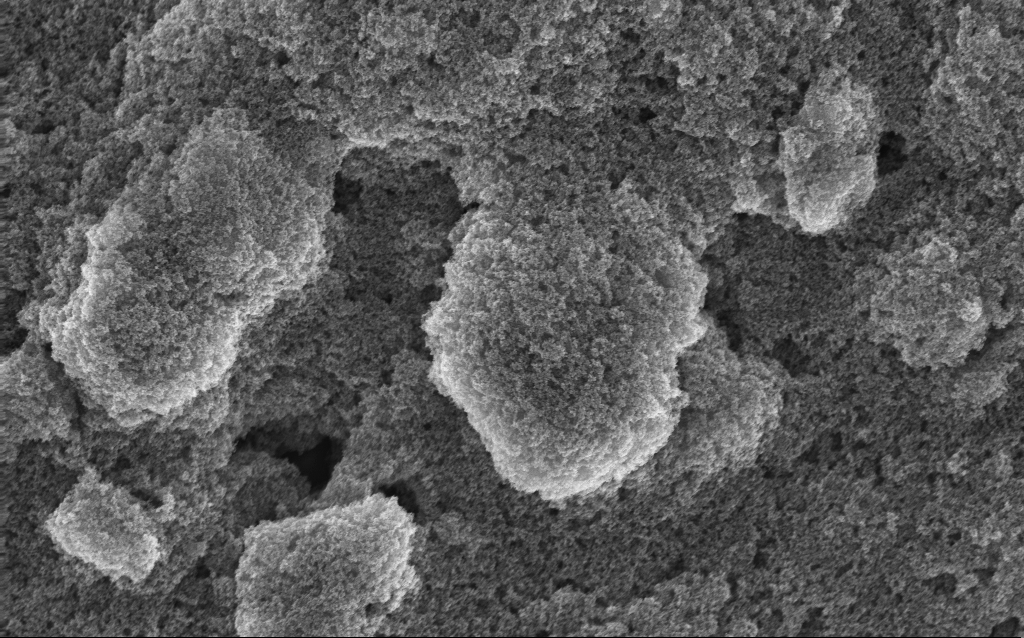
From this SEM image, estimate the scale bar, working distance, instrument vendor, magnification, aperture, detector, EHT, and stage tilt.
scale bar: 1000 nm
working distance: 2.7 mm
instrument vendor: Zeiss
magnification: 20.87 K X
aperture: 20 µm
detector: InLens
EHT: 10 kV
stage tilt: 0°